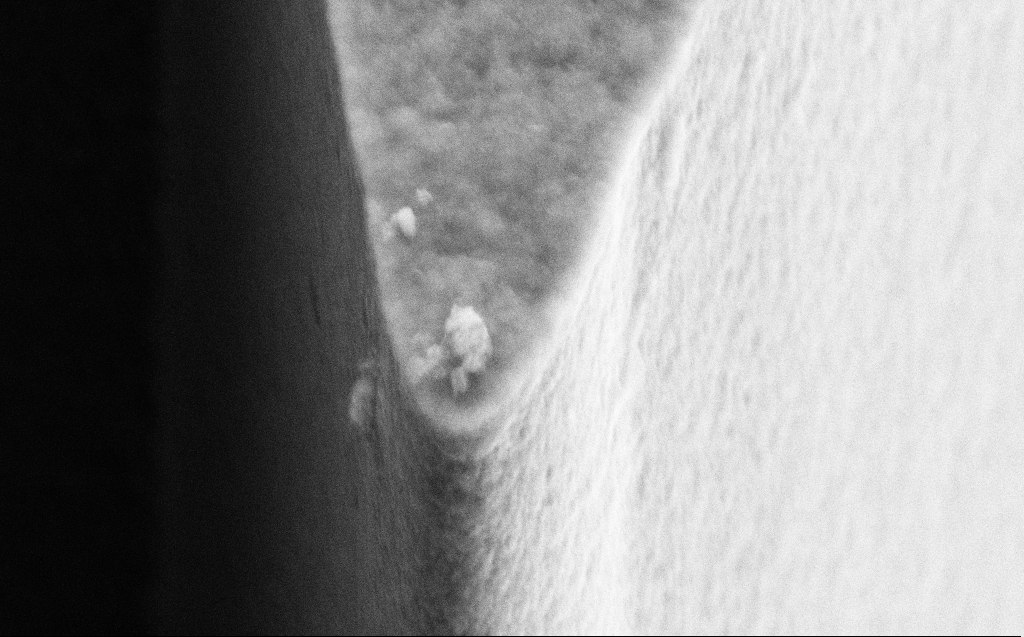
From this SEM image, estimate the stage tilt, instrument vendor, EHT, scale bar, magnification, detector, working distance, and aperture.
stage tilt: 45°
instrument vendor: Zeiss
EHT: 10 kV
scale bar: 1000 nm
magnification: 51.93 K X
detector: SE2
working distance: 6 mm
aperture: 30 µm